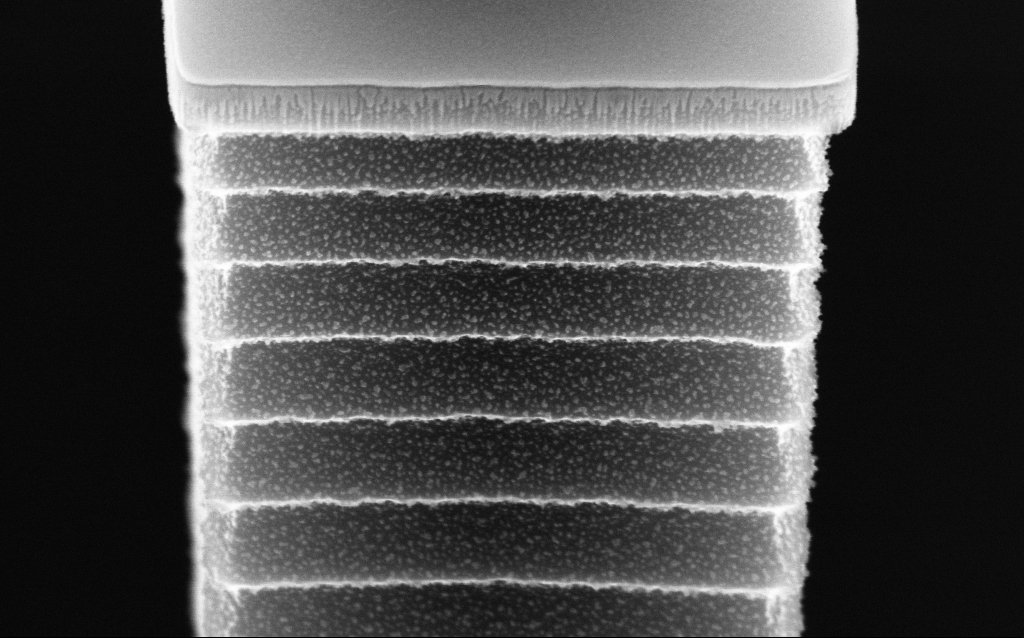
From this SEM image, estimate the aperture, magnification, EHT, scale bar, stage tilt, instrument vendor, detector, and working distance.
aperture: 30 µm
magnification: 125.43 K X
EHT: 10 kV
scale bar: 200 nm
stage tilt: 45°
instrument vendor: Zeiss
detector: InLens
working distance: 4.8 mm